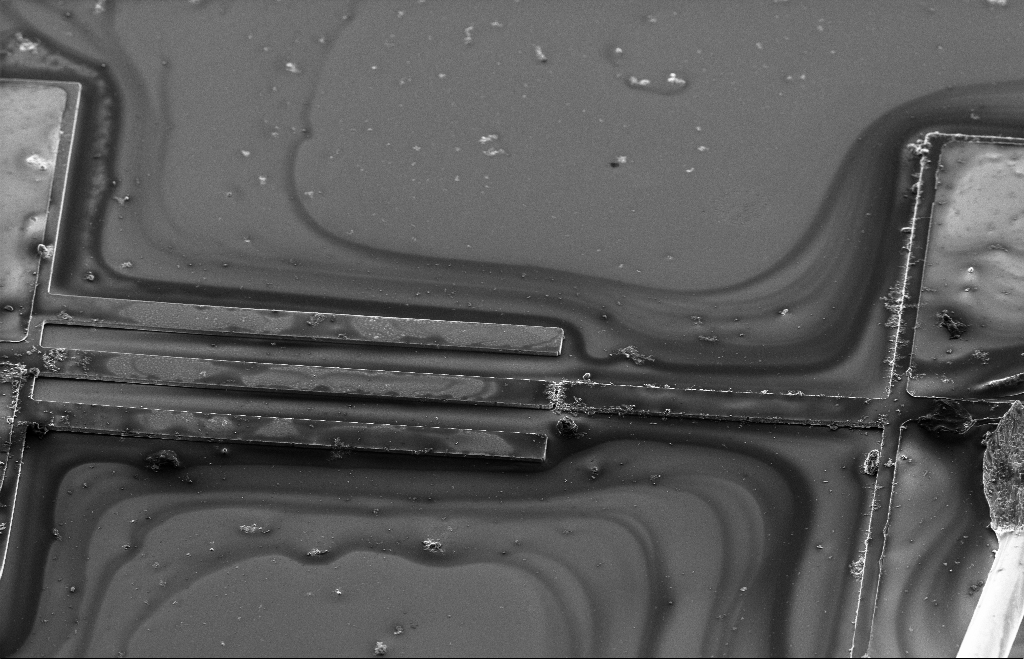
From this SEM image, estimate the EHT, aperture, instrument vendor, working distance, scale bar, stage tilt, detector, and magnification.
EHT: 5 kV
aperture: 20 µm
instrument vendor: Zeiss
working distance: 9 mm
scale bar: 20000 nm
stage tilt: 40.8°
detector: SE2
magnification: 0.699 K X